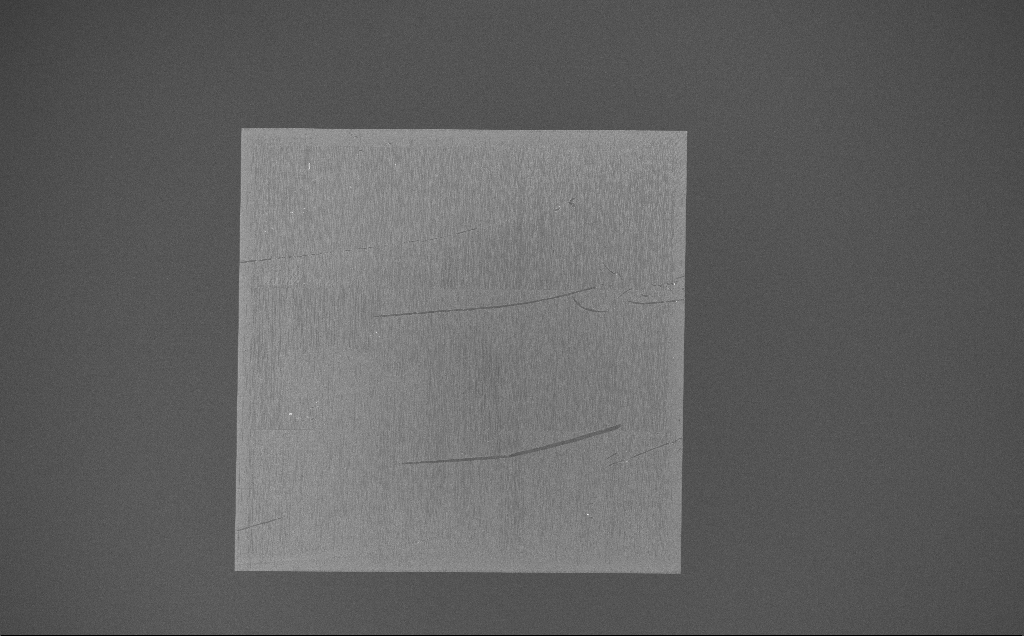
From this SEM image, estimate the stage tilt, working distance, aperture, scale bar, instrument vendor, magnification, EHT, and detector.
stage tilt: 0°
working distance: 7 mm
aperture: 30 µm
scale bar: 200000 nm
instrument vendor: Zeiss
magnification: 0.165 K X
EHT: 10 kV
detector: InLens